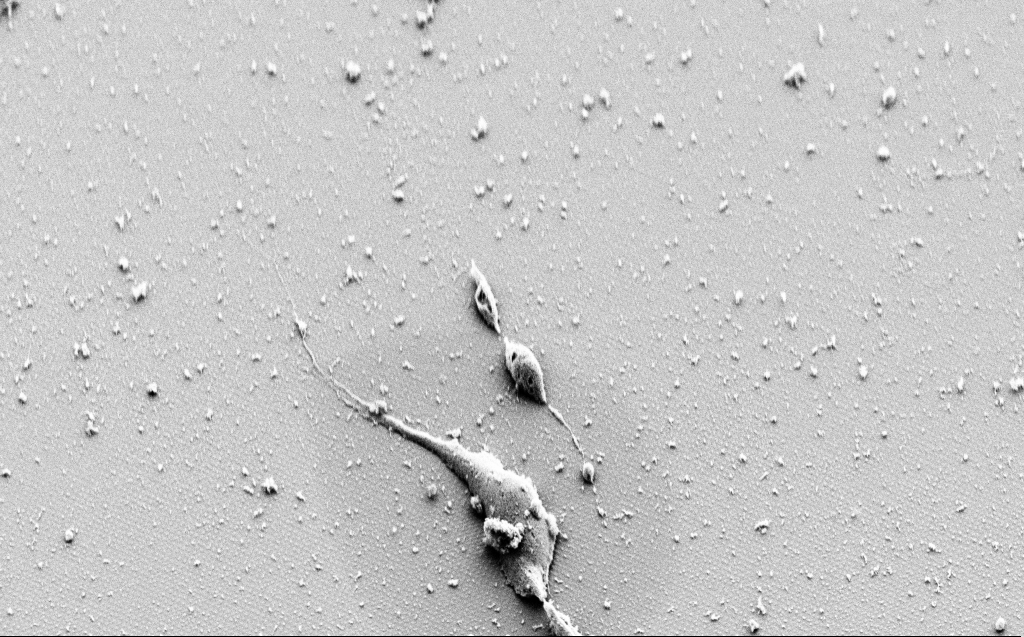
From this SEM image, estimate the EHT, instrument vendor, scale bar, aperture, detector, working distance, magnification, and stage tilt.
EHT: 3 kV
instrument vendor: Zeiss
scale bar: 10000 nm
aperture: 30 µm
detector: SE2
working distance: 9 mm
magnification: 4.13 K X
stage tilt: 45°